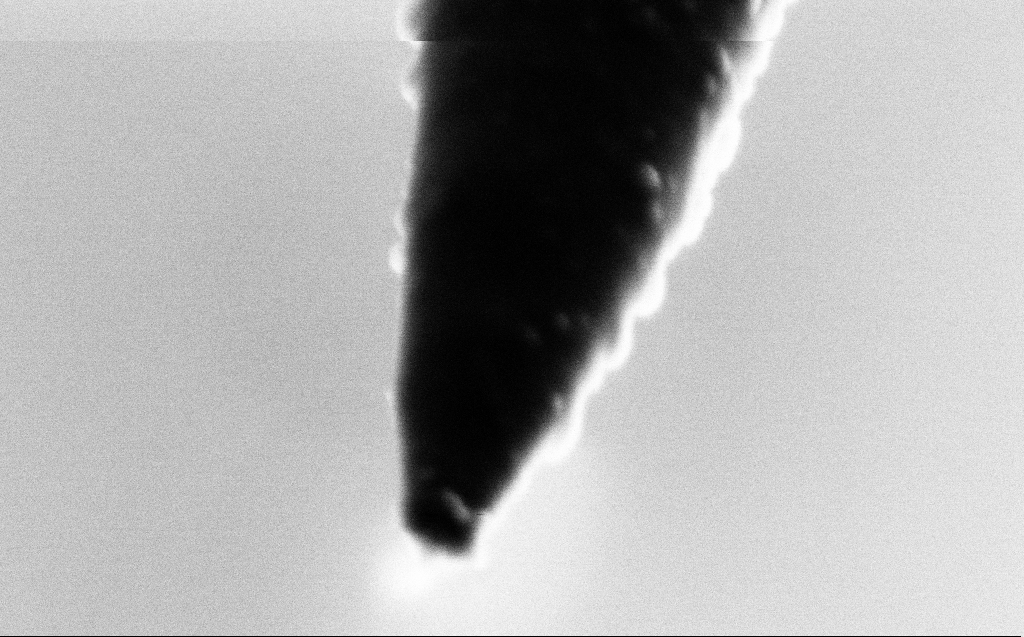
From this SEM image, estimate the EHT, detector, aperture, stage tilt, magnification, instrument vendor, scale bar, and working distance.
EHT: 1.5 kV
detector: SE2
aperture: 30 µm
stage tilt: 45°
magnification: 285.74 K X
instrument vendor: Zeiss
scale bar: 100 nm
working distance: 3 mm